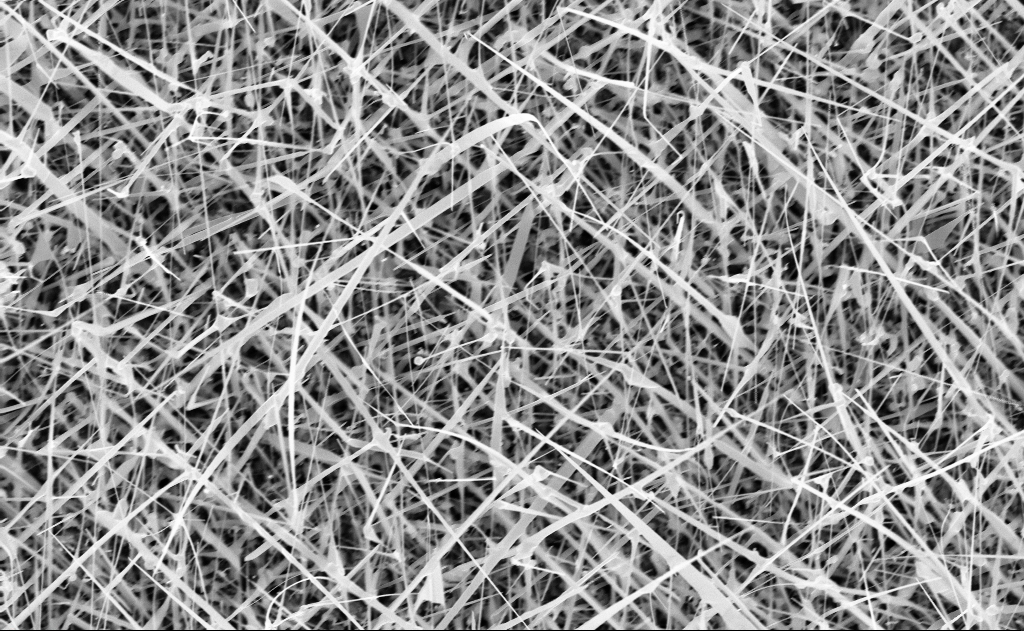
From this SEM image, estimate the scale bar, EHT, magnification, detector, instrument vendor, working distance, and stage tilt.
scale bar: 2000 nm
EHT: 10 kV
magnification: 20 K X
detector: InLens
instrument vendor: Zeiss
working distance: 10 mm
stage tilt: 0°